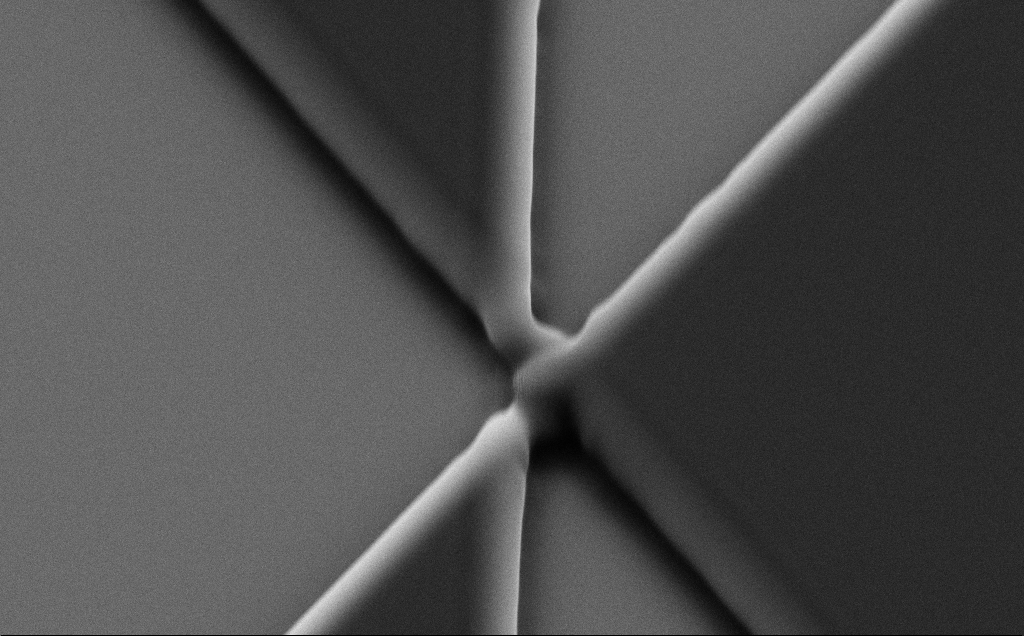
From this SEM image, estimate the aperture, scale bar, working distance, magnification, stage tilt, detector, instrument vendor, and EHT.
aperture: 30 µm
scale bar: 2000 nm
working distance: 8 mm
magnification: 10.46 K X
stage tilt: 35°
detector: SE2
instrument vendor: Zeiss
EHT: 10 kV